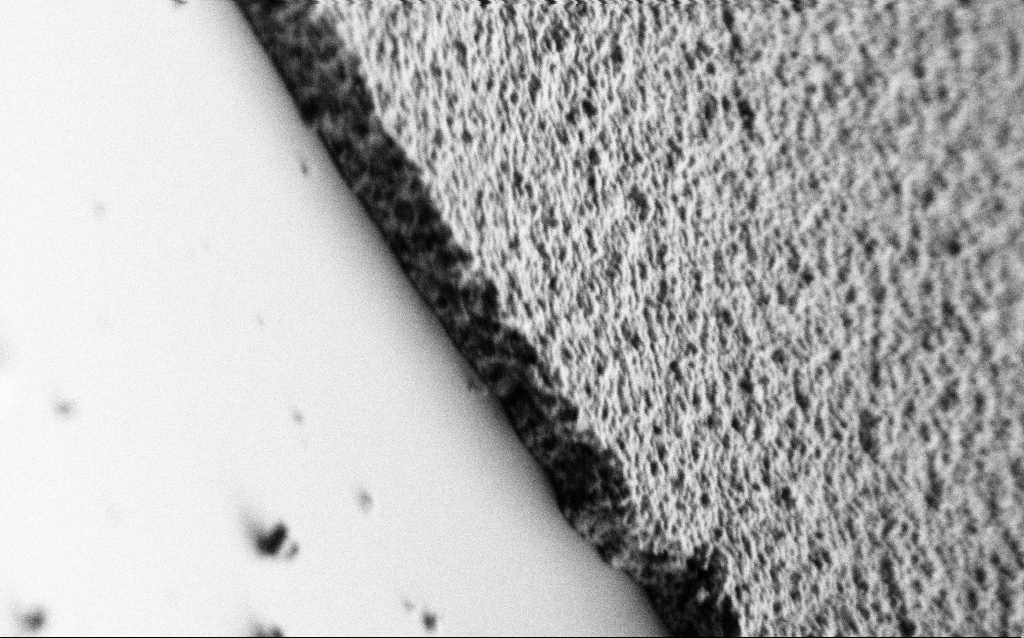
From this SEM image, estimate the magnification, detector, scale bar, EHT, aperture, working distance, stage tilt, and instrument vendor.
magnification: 71.85 K X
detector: SE2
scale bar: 1000 nm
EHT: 1 kV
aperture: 30 µm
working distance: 5 mm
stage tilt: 45°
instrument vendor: Zeiss